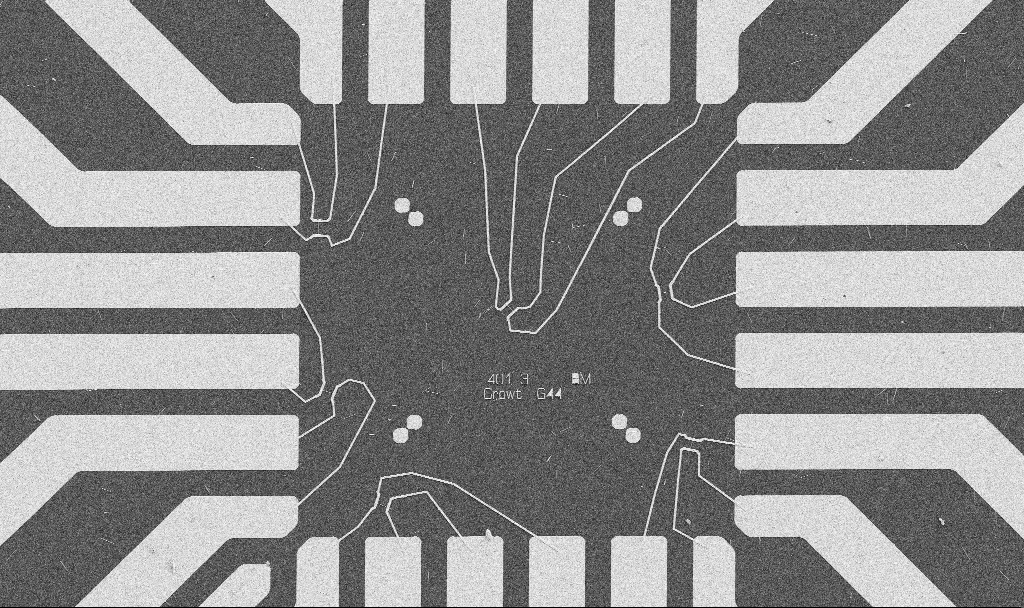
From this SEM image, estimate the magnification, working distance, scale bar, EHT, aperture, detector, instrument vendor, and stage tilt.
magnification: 1 K X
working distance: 10.7 mm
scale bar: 20000 nm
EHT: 5 kV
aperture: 30 µm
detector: SE2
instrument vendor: Zeiss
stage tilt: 0°